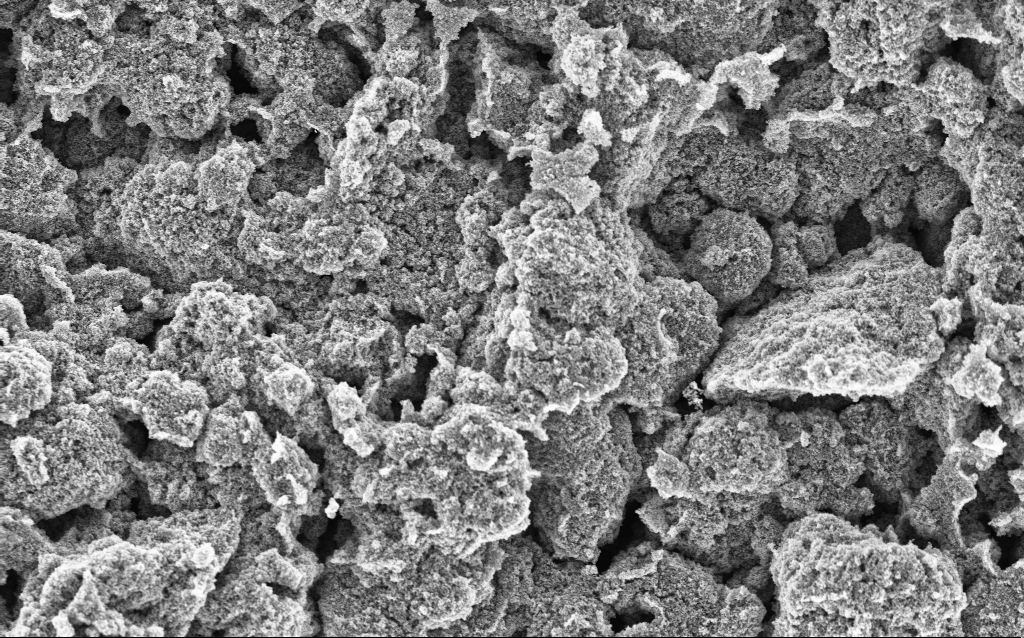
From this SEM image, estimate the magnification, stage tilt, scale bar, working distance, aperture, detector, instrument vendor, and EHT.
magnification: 6.42 K X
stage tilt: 0°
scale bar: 10000 nm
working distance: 4.4 mm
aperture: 30 µm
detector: InLens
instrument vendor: Zeiss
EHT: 5 kV